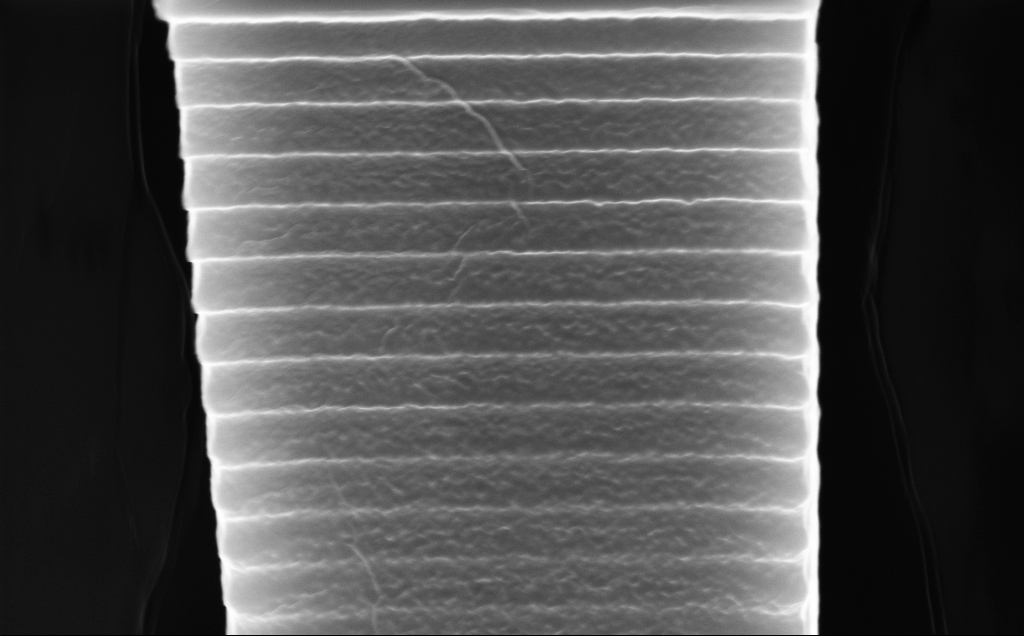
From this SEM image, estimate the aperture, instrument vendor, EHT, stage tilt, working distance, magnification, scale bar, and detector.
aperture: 30 µm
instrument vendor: Zeiss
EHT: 10 kV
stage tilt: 45°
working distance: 5 mm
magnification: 50.89 K X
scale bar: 1000 nm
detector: InLens